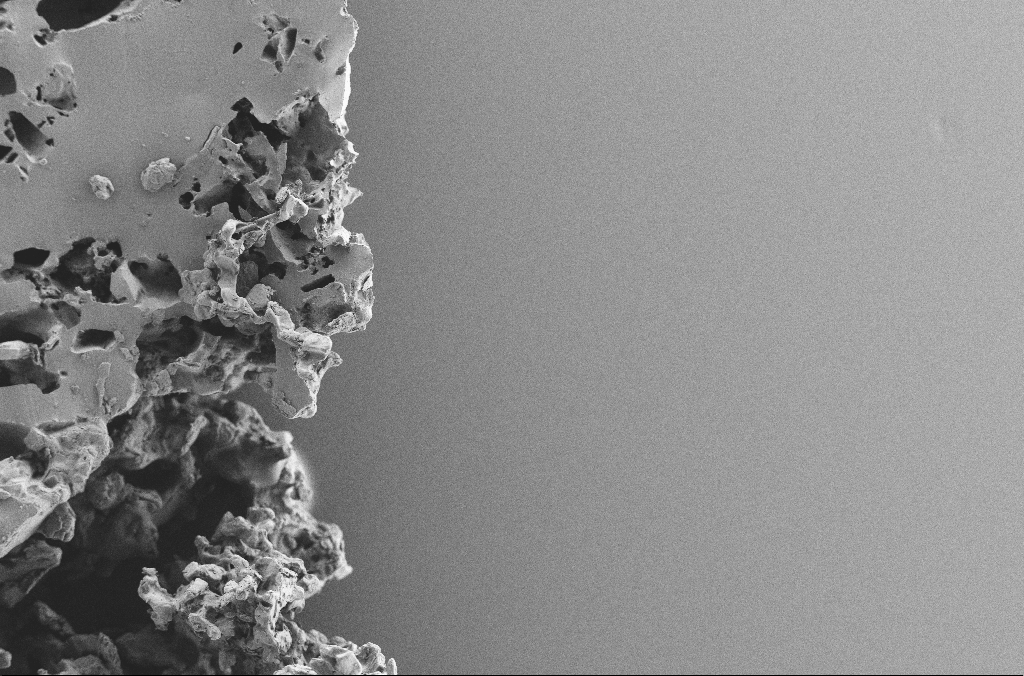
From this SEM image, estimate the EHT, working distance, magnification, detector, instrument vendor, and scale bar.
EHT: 2 kV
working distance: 3.1 mm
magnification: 0.25 K X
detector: SE2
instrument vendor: Zeiss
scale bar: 100000 nm